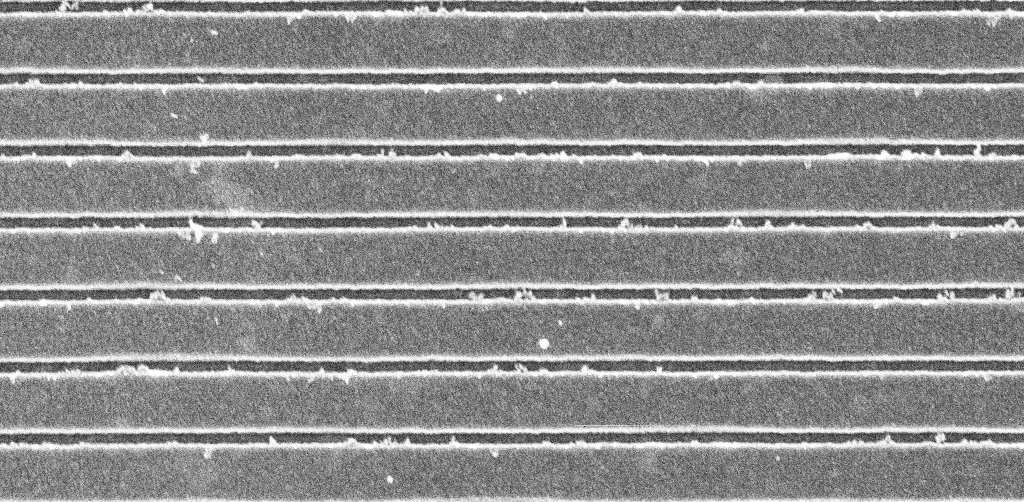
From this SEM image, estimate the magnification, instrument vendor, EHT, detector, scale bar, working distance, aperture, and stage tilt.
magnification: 44.3 K X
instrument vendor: Zeiss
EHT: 5 kV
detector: InLens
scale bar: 1000 nm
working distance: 5.2 mm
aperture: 30 µm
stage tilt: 0°